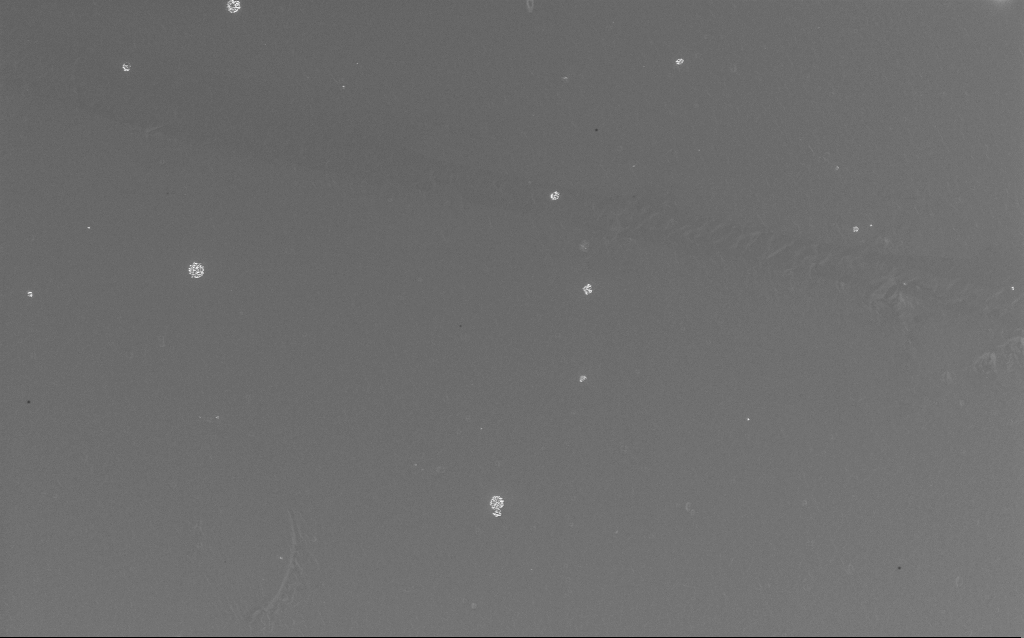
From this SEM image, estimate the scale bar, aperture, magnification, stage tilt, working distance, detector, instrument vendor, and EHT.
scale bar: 10000 nm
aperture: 30 µm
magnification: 2.26 K X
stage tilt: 0°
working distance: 2 mm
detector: InLens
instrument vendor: Zeiss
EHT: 10 kV